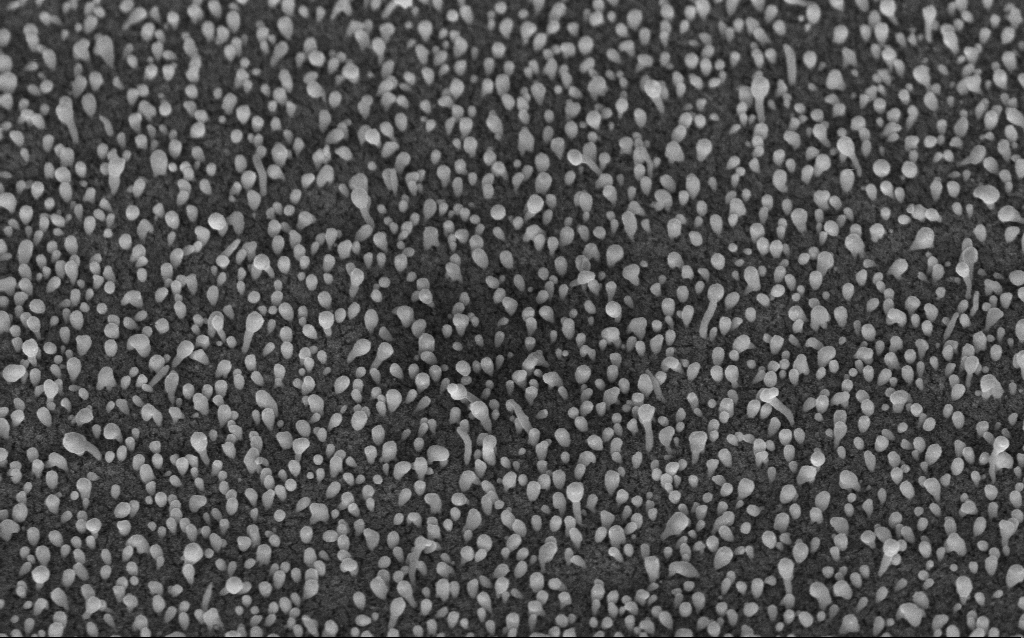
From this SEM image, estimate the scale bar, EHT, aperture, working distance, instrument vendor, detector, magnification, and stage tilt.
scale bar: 1000 nm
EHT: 5 kV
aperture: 30 µm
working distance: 6.2 mm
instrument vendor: Zeiss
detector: InLens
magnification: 50 K X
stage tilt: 45°